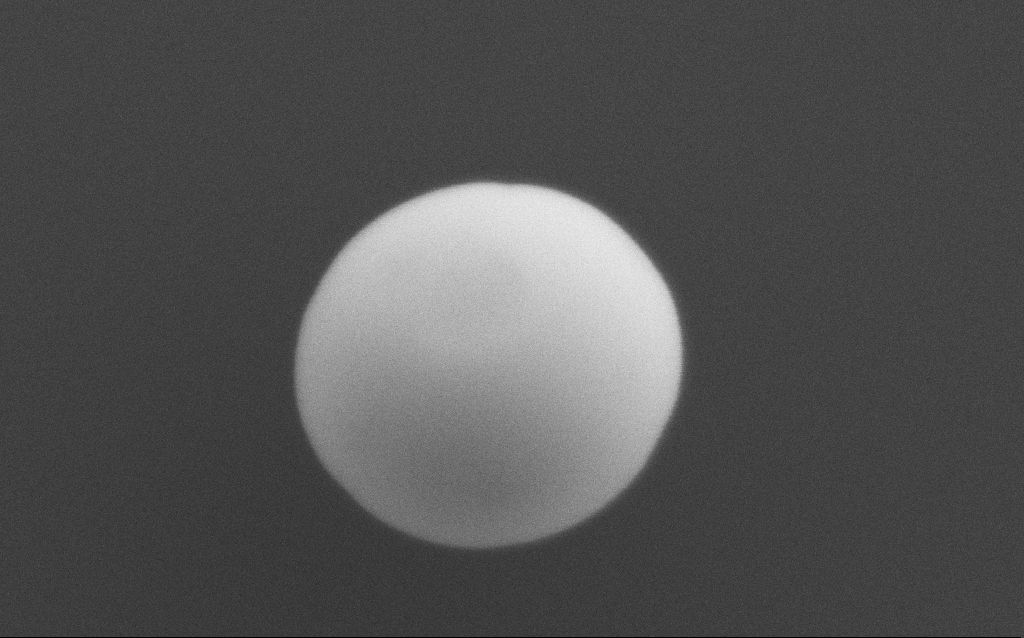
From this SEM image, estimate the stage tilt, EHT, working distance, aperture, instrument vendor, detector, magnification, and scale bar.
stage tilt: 0°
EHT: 10 kV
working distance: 4 mm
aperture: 30 µm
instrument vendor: Zeiss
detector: SE2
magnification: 179.34 K X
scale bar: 200 nm